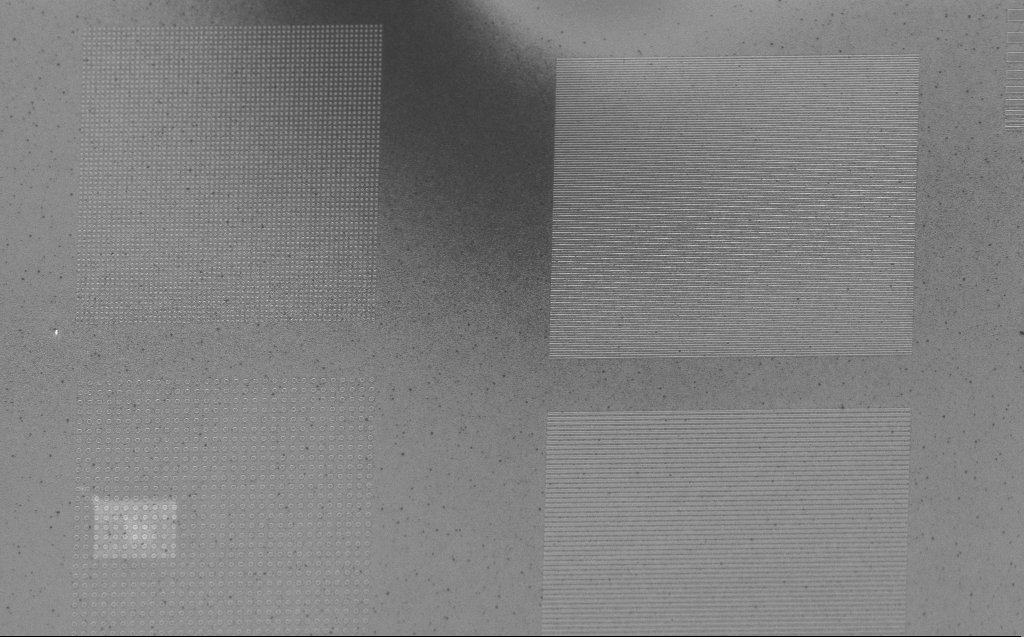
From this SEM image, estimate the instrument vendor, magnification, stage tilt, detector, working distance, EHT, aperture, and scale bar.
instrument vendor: Zeiss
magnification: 4.52 K X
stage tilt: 30°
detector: InLens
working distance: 4 mm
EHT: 5 kV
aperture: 30 µm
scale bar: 10000 nm